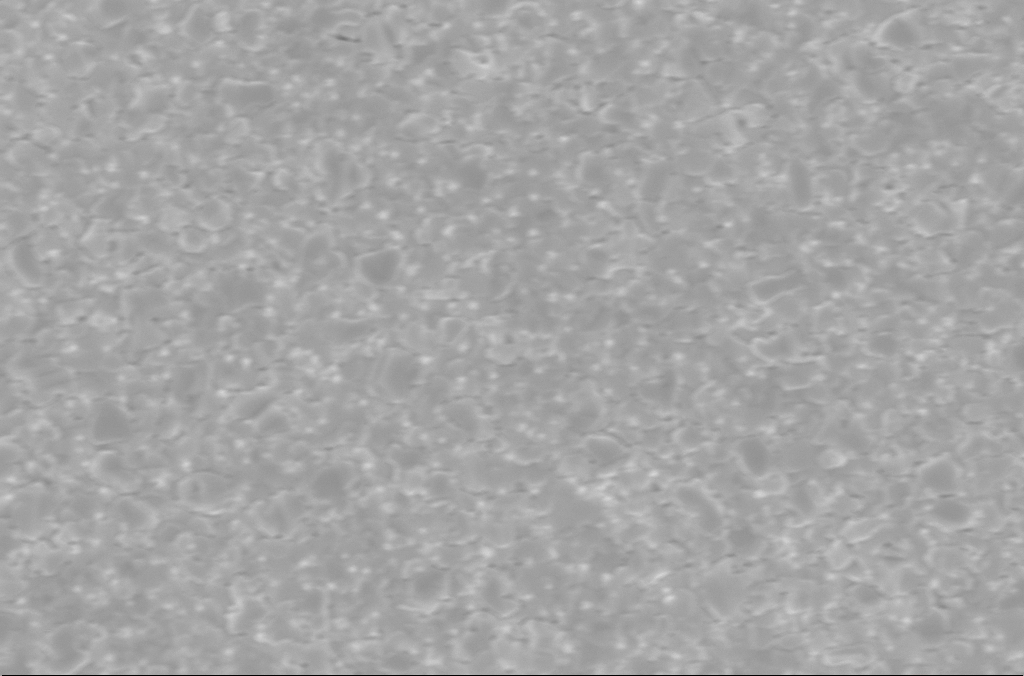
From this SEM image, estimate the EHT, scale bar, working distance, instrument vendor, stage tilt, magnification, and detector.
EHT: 5 kV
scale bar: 2000 nm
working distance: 3 mm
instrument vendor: Zeiss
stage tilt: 0°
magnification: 30 K X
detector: InLens